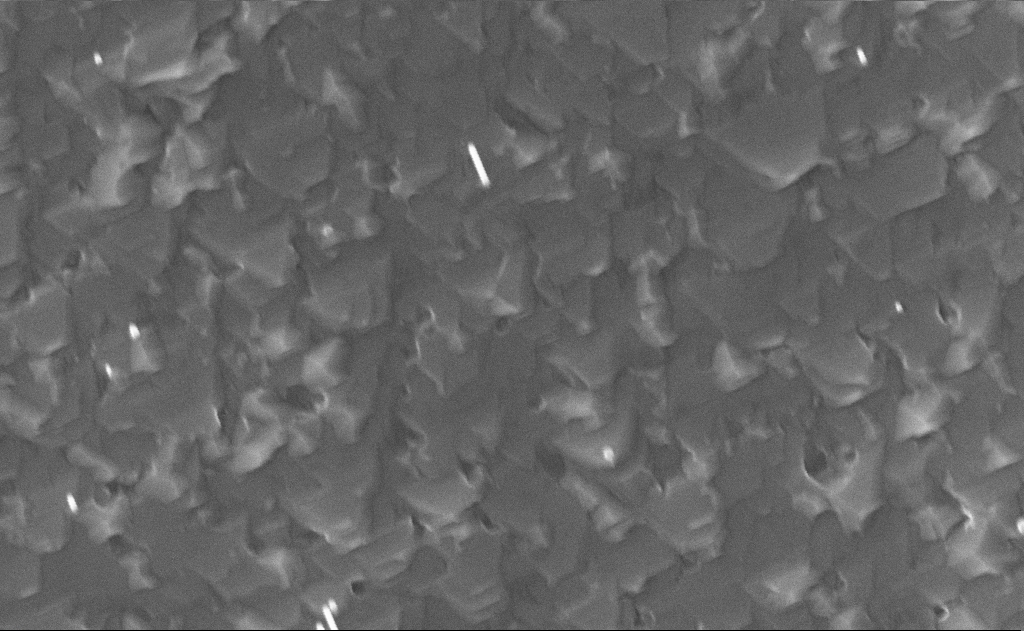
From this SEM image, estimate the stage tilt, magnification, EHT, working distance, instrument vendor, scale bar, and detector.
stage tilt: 0°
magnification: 60 K X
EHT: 10 kV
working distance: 14 mm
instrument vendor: Zeiss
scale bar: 1000 nm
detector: InLens